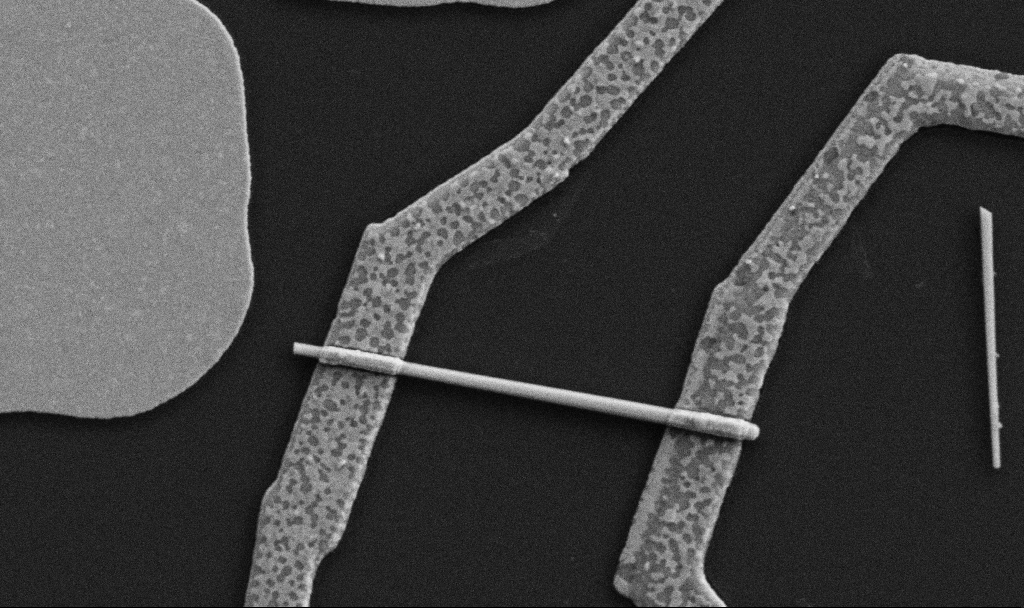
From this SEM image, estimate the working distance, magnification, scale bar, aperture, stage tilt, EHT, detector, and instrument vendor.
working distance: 8.7 mm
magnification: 30 K X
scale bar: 1000 nm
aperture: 30 µm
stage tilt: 0°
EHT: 5 kV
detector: SE2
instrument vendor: Zeiss